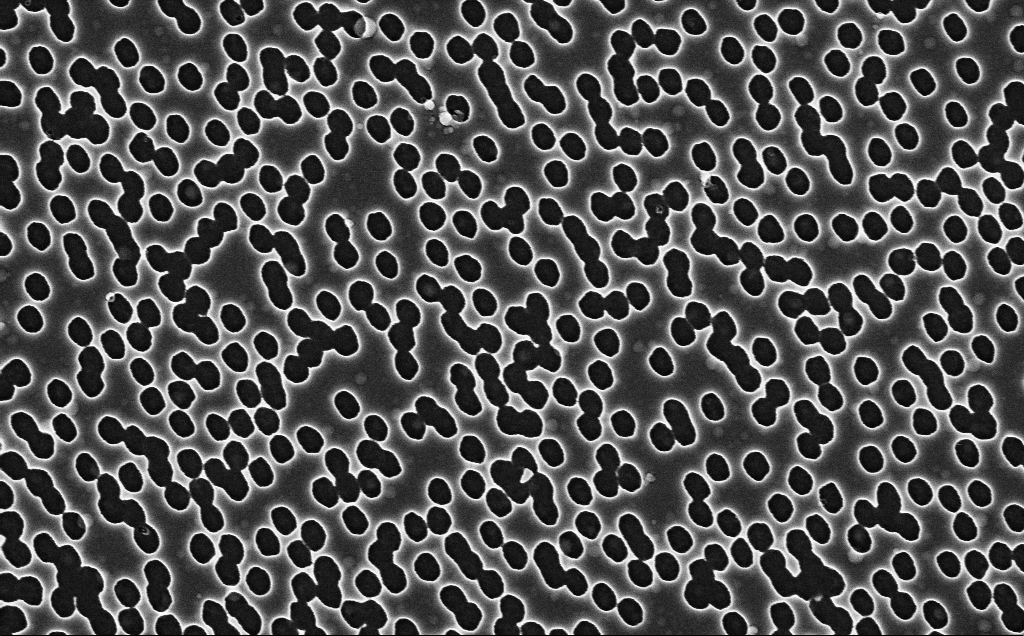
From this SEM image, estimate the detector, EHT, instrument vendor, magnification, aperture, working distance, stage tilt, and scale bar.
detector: InLens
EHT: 3 kV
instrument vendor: Zeiss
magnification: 40 K X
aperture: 30 µm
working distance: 2.4 mm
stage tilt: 0°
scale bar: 1000 nm